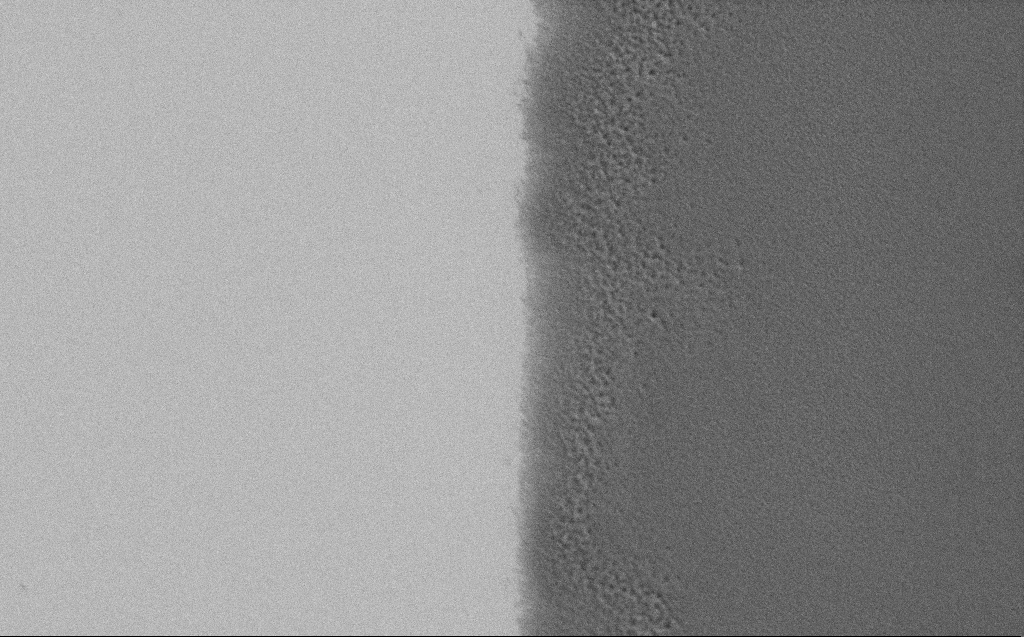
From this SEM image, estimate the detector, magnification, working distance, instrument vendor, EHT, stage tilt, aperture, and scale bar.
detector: SE2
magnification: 16.96 K X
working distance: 6 mm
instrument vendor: Zeiss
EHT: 1.2 kV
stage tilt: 0°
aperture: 30 µm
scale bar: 2000 nm